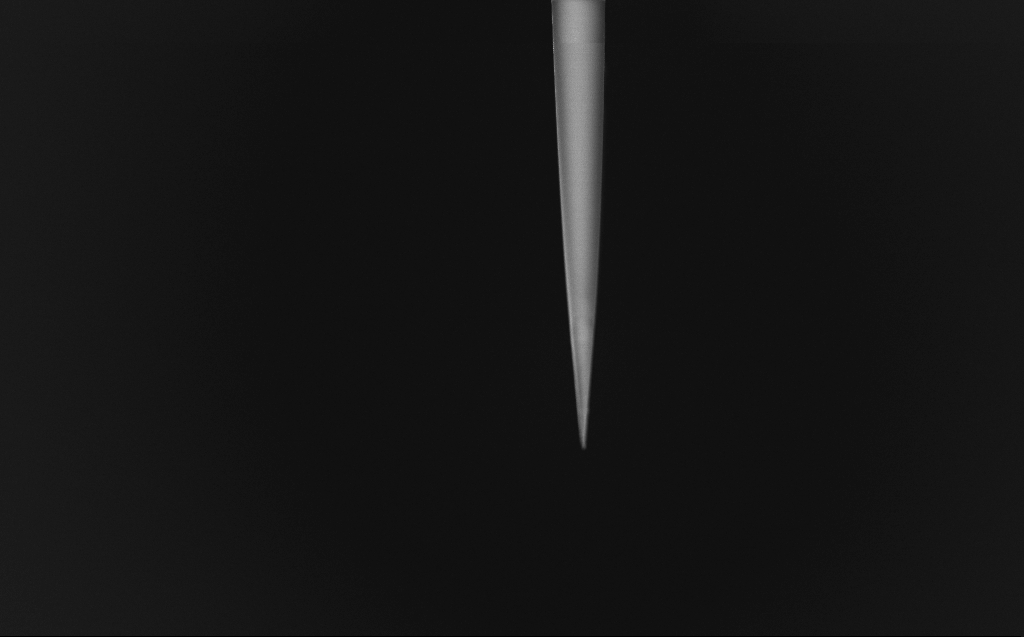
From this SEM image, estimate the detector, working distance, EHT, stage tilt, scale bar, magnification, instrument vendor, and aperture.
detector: InLens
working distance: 5 mm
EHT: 1 kV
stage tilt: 45°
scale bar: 20000 nm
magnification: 1 K X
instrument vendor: Zeiss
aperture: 30 µm